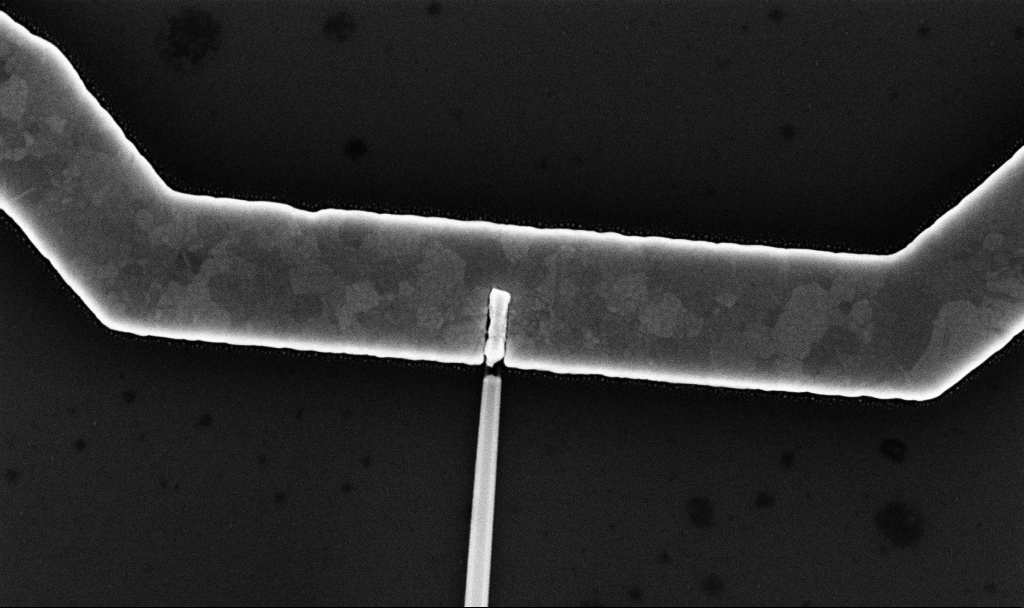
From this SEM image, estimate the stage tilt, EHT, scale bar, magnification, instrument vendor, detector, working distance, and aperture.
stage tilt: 0°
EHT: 10 kV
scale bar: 1000 nm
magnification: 57.2 K X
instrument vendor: Zeiss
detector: InLens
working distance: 7.7 mm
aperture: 30 µm